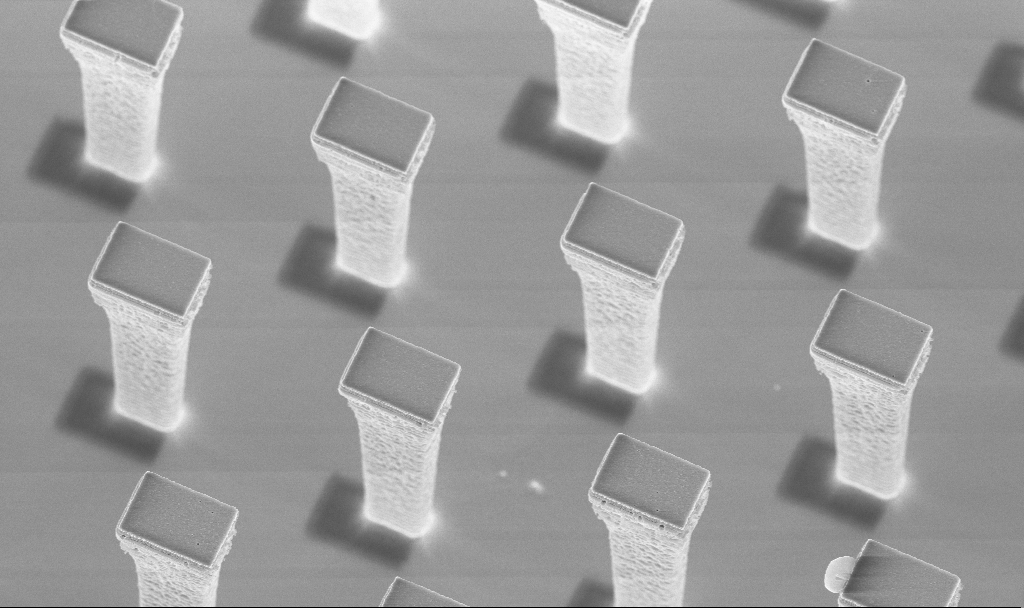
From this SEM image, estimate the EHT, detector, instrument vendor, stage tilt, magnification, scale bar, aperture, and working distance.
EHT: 5 kV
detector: InLens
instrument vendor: Zeiss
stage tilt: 20°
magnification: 8.44 K X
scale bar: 2000 nm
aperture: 30 µm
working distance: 4.3 mm